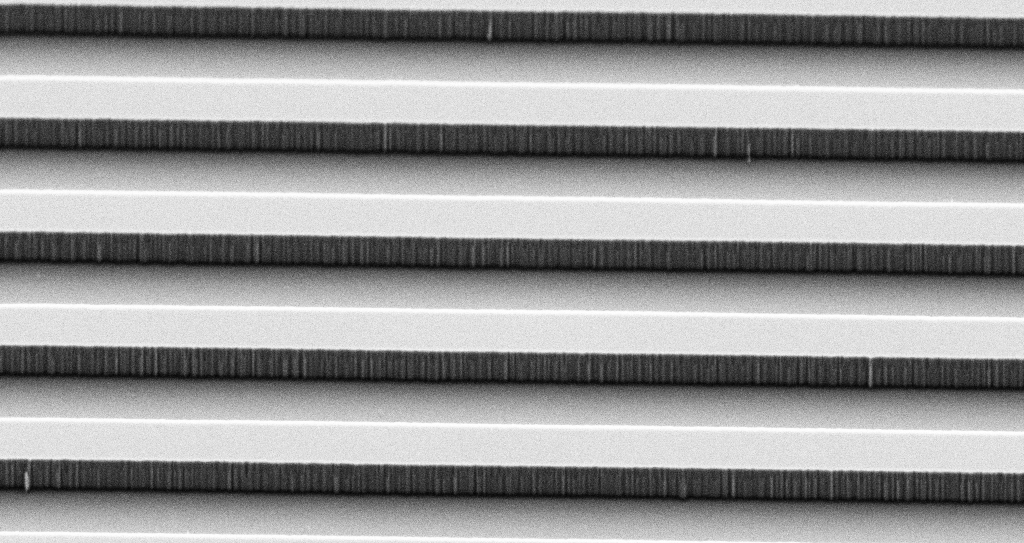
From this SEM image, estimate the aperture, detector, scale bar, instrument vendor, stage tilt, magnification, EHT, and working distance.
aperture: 30 µm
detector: SE2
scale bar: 2000 nm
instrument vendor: Zeiss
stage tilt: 45°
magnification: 10.68 K X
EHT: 3 kV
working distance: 9 mm